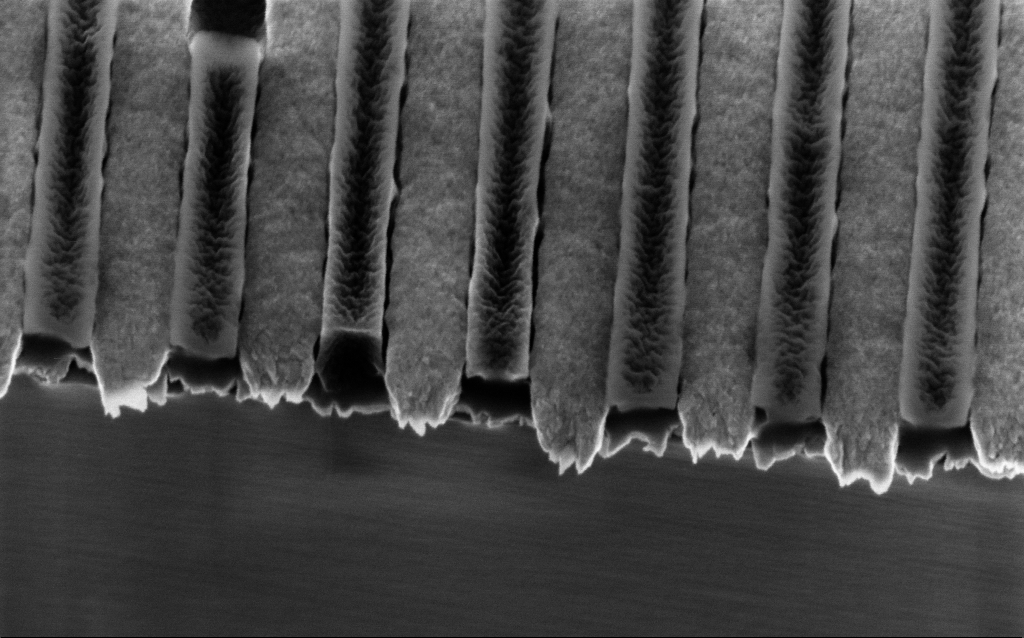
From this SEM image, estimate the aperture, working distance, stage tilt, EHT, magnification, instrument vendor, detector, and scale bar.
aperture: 30 µm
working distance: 4.2 mm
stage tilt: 32°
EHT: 2 kV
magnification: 108.32 K X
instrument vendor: Zeiss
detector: InLens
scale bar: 200 nm